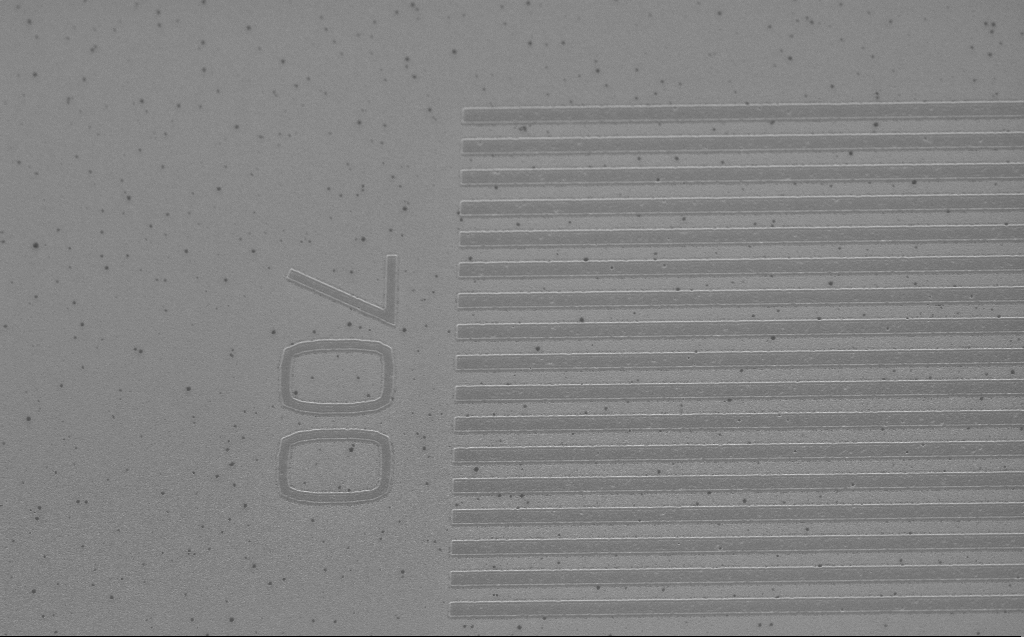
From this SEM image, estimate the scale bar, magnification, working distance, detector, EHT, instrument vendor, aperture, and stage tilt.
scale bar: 2000 nm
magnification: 8.27 K X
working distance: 4 mm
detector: InLens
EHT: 5 kV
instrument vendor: Zeiss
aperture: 30 µm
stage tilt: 30°